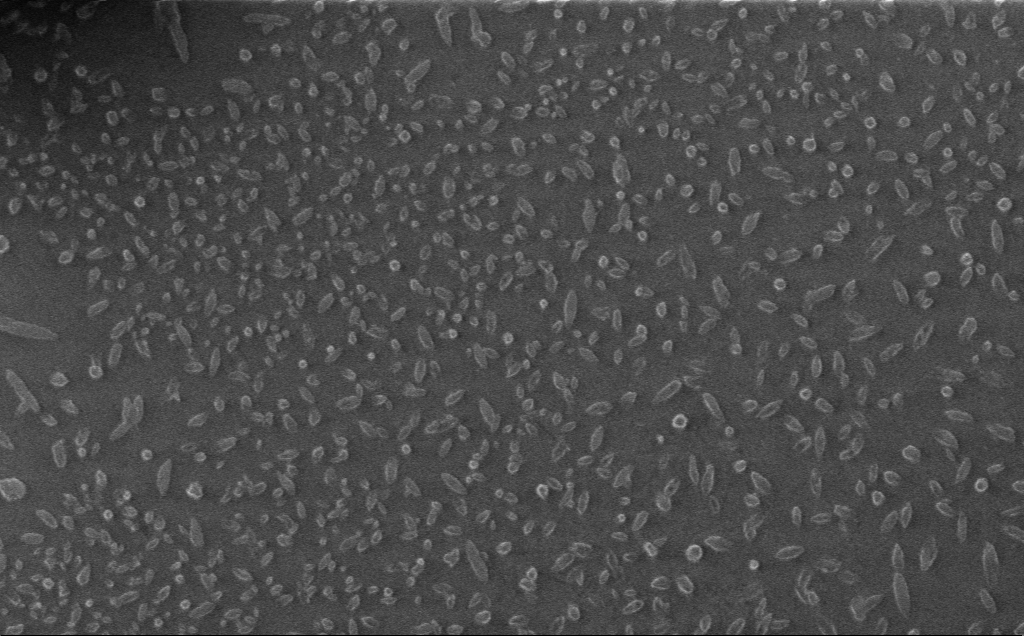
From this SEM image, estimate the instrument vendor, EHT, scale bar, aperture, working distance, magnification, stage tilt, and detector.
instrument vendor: Zeiss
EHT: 1 kV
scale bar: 2000 nm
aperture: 30 µm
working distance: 3 mm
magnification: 8.23 K X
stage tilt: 0°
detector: InLens